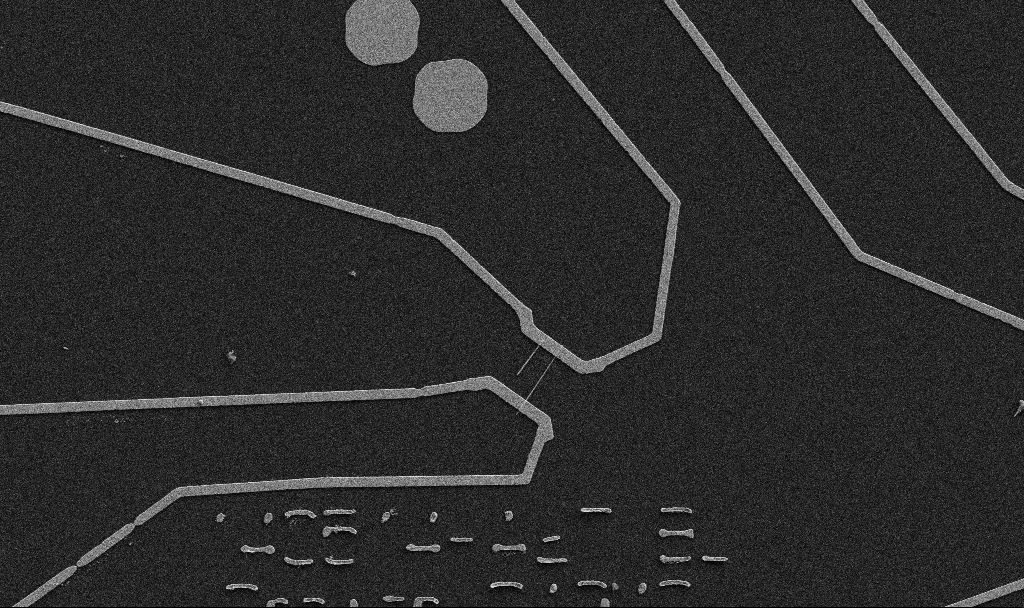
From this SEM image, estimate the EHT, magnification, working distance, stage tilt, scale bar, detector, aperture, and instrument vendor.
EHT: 5 kV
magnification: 5 K X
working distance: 10.7 mm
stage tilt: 0°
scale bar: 10000 nm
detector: SE2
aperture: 30 µm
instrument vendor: Zeiss